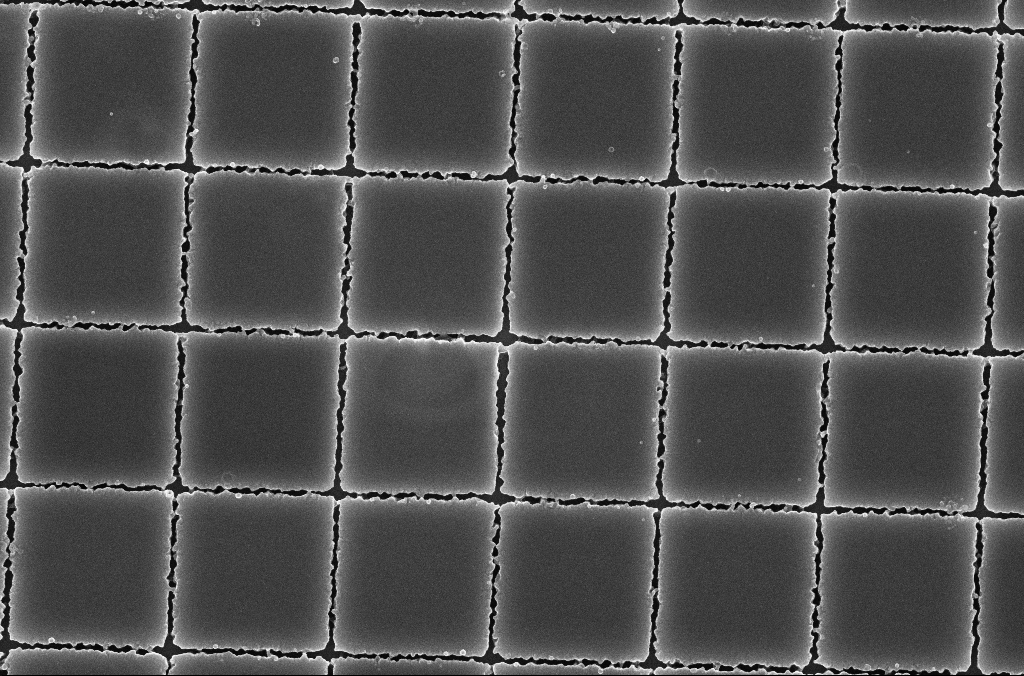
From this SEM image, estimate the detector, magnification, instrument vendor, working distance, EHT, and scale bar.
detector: InLens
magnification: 29.98 K X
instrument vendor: Zeiss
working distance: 4.2 mm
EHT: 5 kV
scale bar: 1000 nm